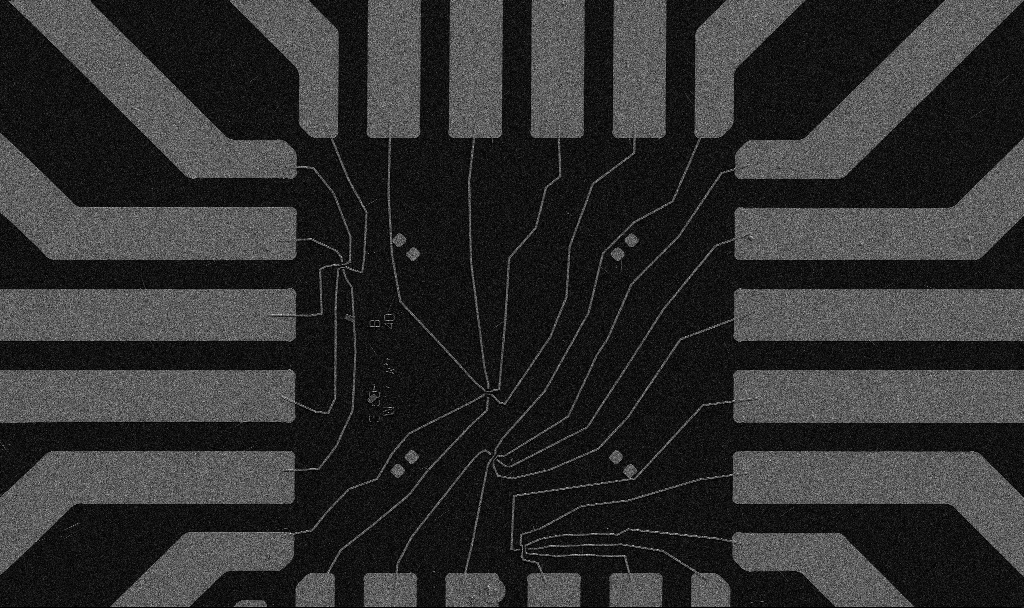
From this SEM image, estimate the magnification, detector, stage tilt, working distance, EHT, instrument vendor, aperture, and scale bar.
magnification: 1 K X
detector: SE2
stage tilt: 0°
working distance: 10.7 mm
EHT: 5 kV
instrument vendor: Zeiss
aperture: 30 µm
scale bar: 20000 nm